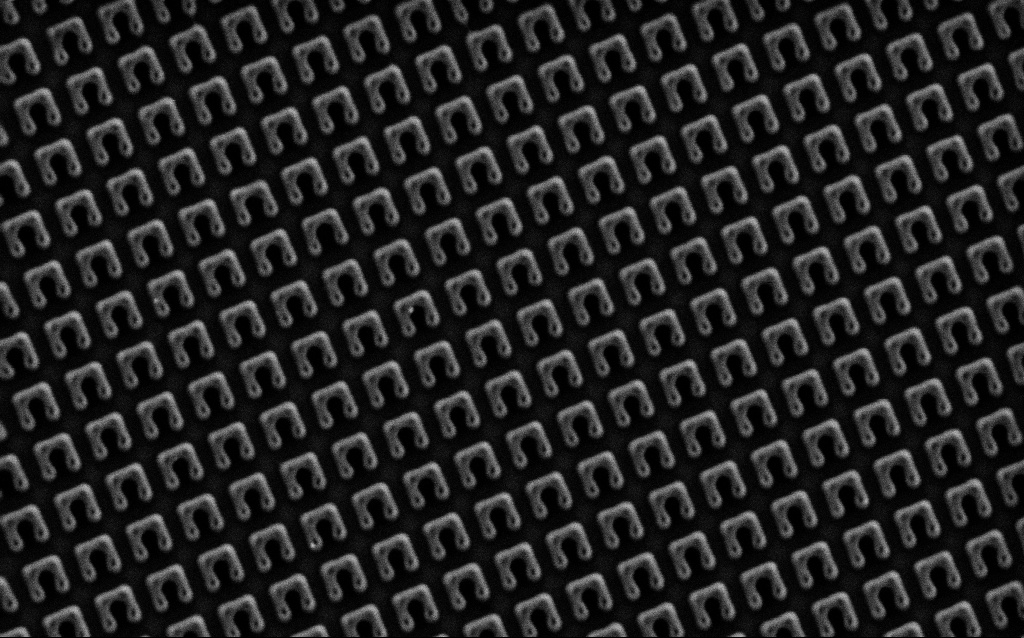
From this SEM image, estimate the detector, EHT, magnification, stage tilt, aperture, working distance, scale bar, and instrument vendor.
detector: SE2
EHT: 1.5 kV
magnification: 43.42 K X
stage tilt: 0°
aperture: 30 µm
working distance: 7.9 mm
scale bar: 1000 nm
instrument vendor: Zeiss